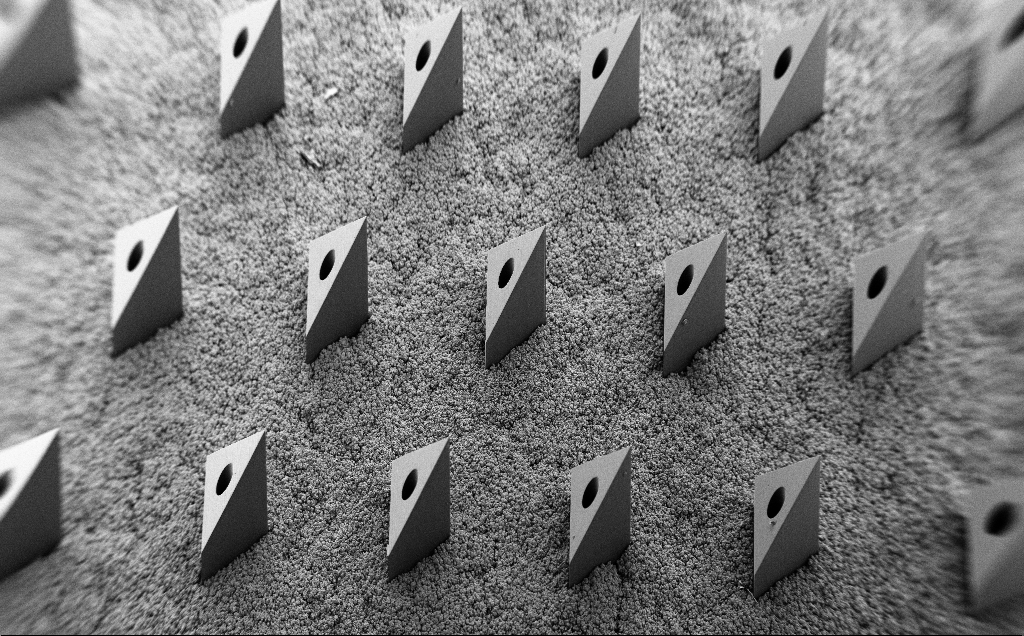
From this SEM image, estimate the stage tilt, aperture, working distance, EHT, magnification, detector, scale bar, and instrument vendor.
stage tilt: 35°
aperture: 30 µm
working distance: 9 mm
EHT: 5 kV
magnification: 0.067 K X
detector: SE2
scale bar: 1e+06 nm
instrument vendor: Zeiss